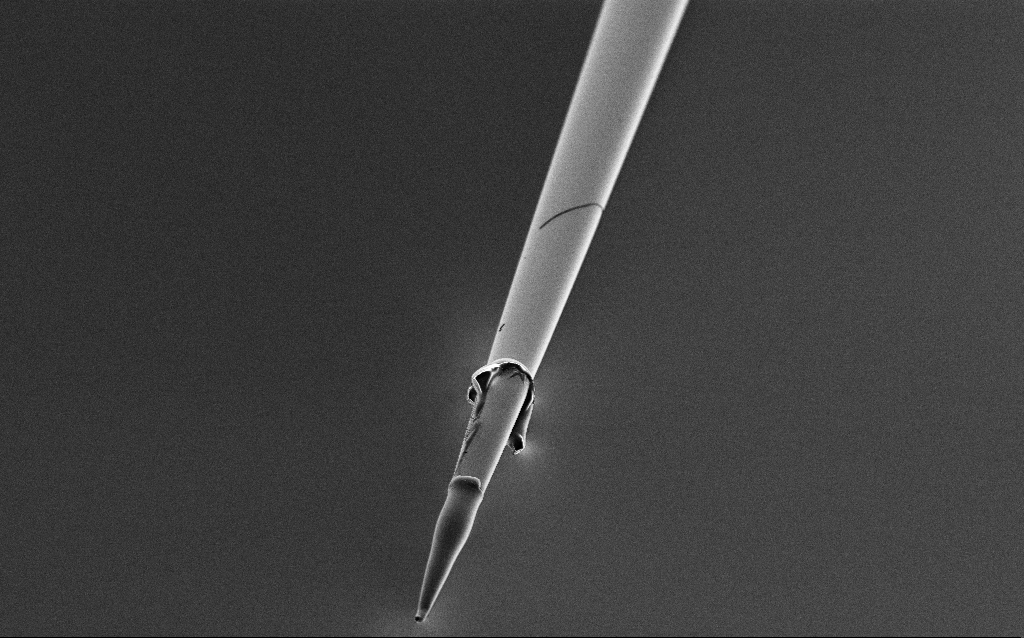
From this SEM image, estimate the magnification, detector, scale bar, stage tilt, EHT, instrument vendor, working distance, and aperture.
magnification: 1 K X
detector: SE2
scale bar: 20000 nm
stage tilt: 45°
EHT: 1 kV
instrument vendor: Zeiss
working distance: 6.1 mm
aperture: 30 µm